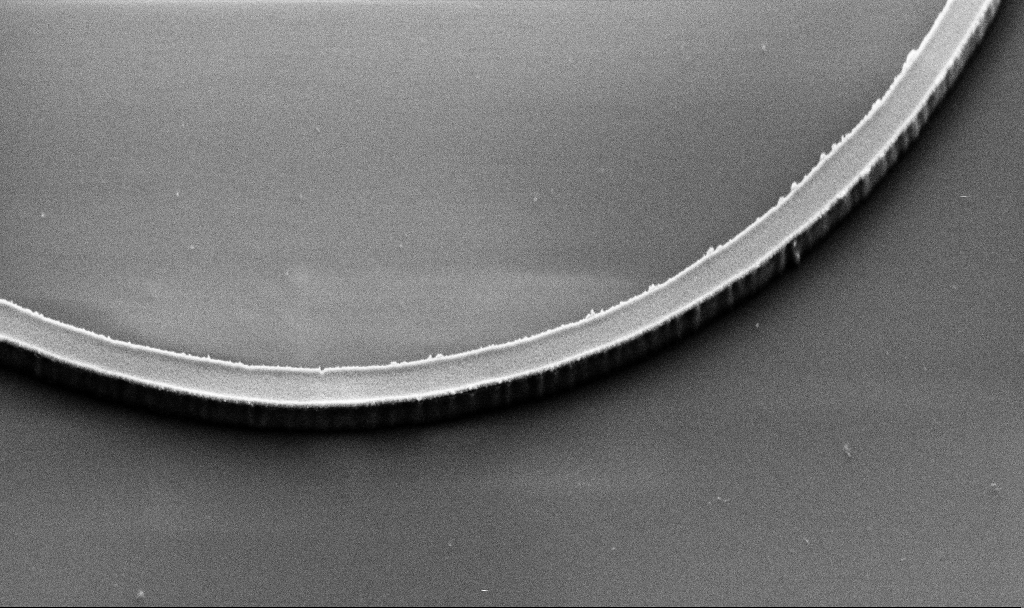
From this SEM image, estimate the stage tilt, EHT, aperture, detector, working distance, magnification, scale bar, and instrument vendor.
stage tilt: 45°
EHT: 3 kV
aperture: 30 µm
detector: SE2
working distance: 7.2 mm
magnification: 27.93 K X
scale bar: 1000 nm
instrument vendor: Zeiss